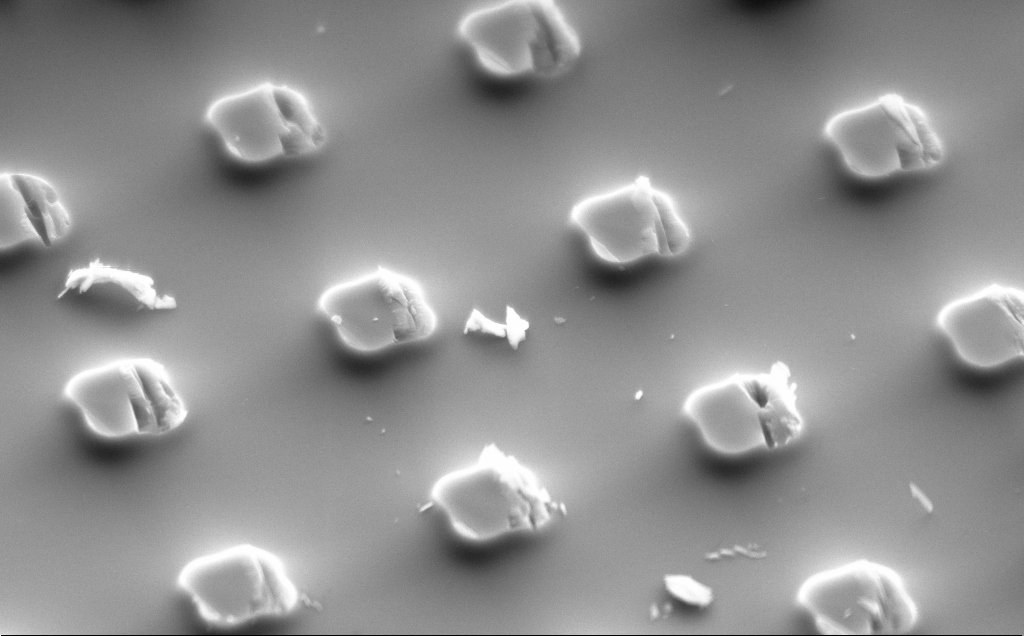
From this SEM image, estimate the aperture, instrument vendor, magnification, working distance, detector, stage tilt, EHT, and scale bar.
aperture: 120 µm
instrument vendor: Zeiss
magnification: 10.17 K X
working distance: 10 mm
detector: SE2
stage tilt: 48.7°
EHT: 10 kV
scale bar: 2000 nm